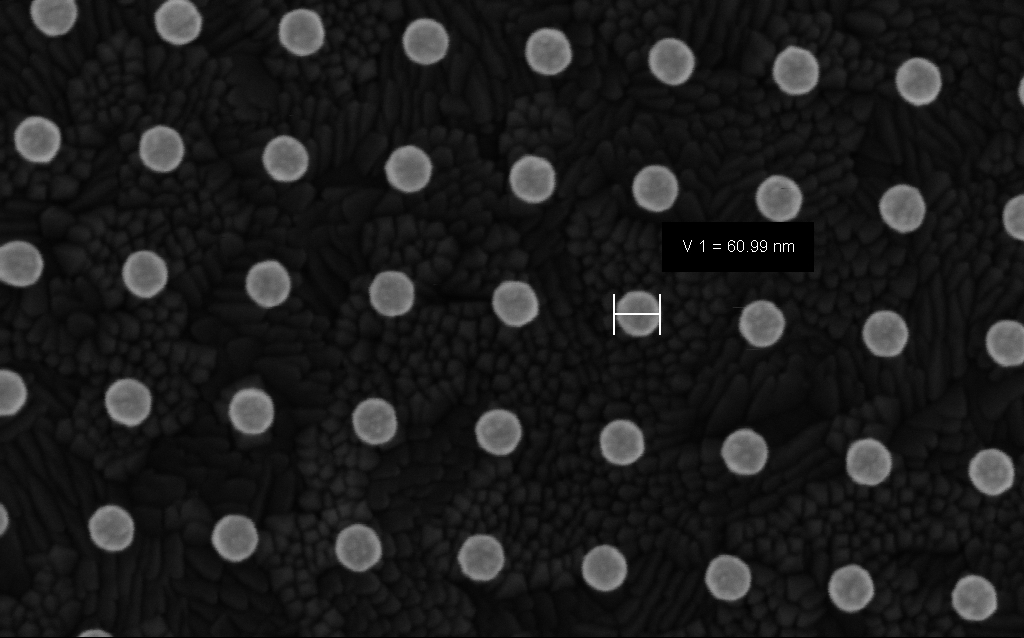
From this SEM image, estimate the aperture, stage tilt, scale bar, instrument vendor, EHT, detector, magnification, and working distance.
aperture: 30 µm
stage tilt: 0°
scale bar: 200 nm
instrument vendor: Zeiss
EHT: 5 kV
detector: InLens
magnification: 276.93 K X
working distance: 3.7 mm